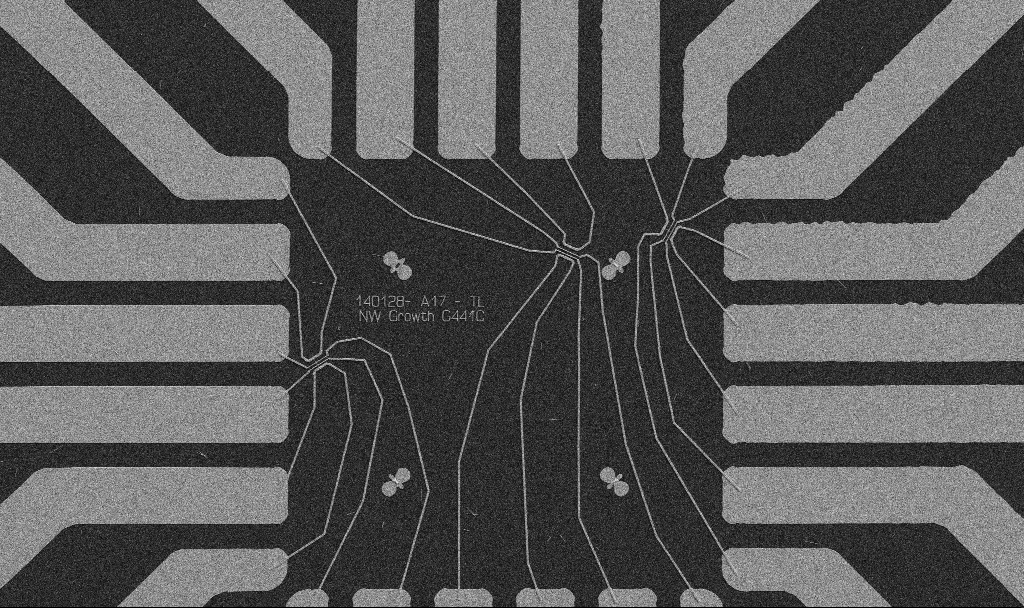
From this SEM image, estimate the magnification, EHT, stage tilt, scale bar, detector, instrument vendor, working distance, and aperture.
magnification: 1 K X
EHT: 5 kV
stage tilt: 0°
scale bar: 20000 nm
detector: SE2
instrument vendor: Zeiss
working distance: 10.7 mm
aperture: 30 µm